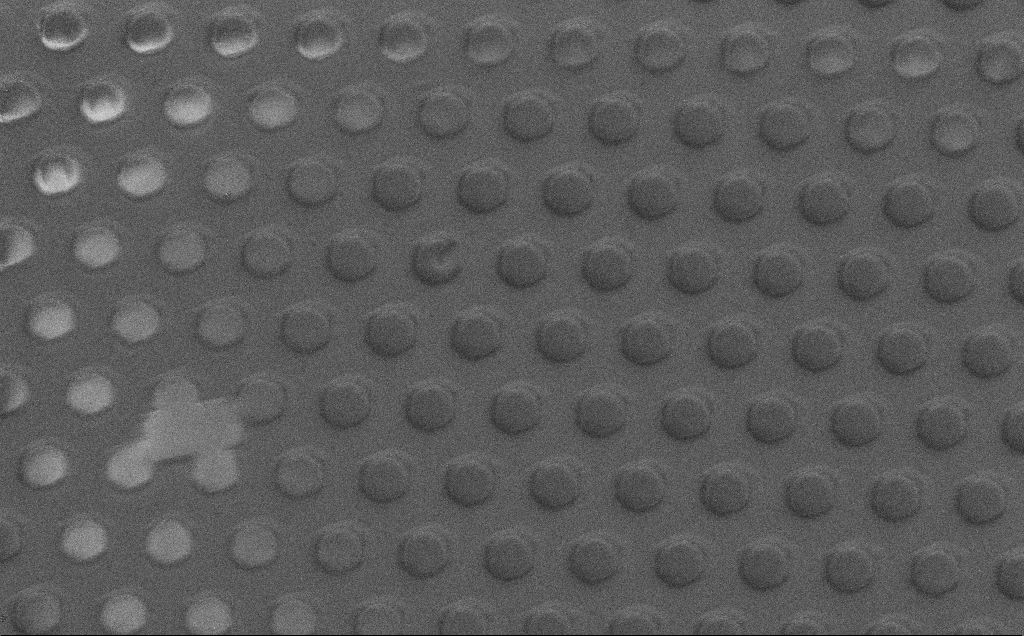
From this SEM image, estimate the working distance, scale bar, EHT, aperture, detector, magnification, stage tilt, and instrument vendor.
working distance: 5 mm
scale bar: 20000 nm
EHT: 1.5 kV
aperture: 30 µm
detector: SE2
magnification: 1.57 K X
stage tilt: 0°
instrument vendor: Zeiss